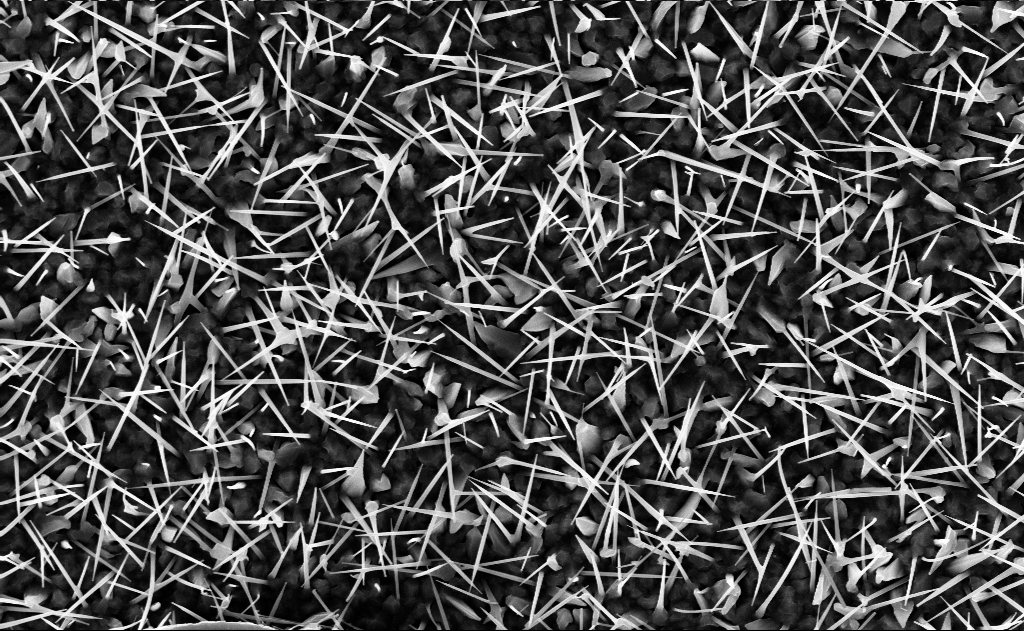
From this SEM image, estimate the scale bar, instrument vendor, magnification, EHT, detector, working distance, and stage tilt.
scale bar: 2000 nm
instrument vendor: Zeiss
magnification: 20 K X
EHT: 10 kV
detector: InLens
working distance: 9 mm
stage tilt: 0°